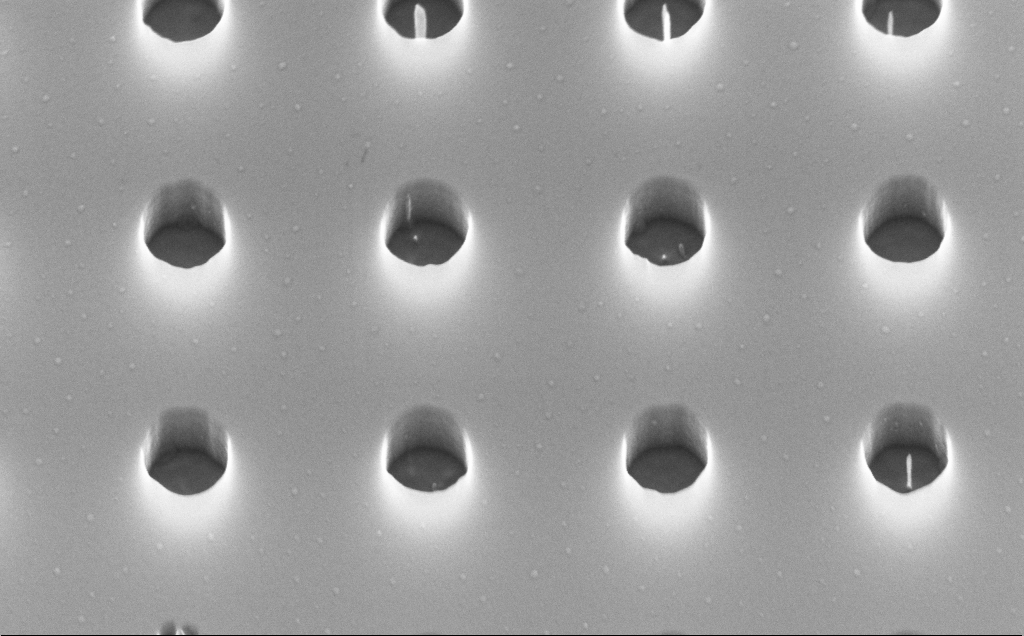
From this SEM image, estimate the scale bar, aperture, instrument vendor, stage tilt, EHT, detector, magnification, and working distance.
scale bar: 200 nm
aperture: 30 µm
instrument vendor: Zeiss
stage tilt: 45°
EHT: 10 kV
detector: InLens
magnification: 84.27 K X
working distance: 4 mm